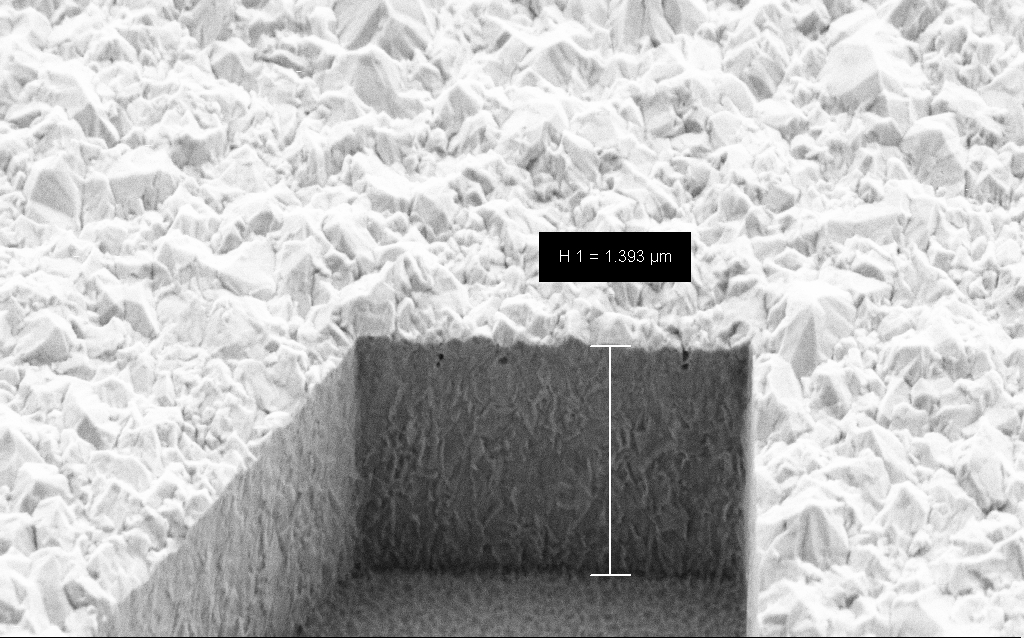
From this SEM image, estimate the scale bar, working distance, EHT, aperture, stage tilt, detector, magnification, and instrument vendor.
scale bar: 1000 nm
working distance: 6.4 mm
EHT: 3 kV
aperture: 30 µm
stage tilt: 45°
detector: SE2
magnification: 60.38 K X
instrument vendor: Zeiss